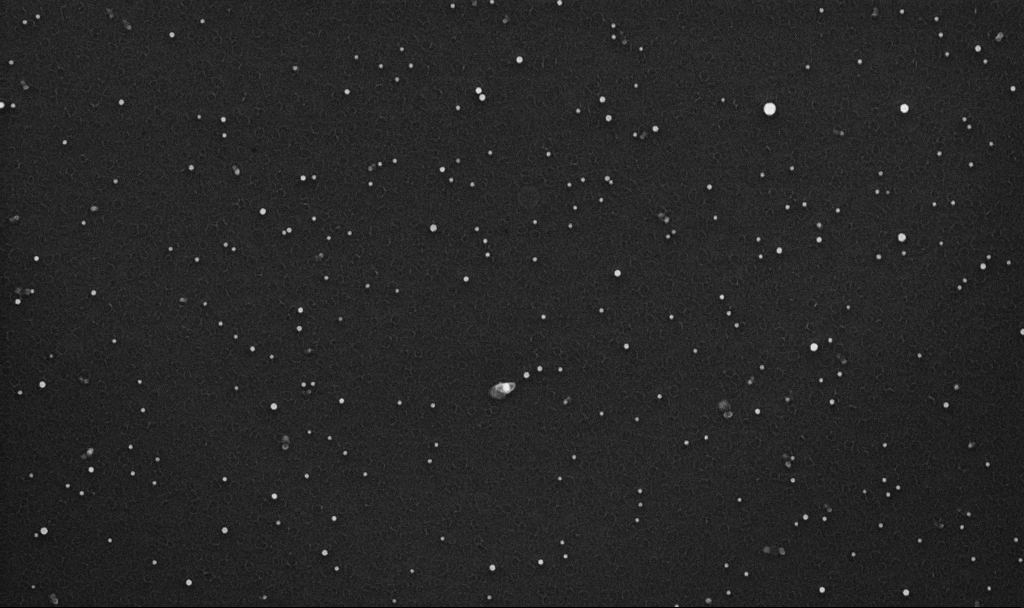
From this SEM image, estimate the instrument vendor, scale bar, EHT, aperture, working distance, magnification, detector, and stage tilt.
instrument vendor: Zeiss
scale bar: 1000 nm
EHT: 10 kV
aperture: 30 µm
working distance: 3.3 mm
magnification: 70 K X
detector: InLens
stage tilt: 0°